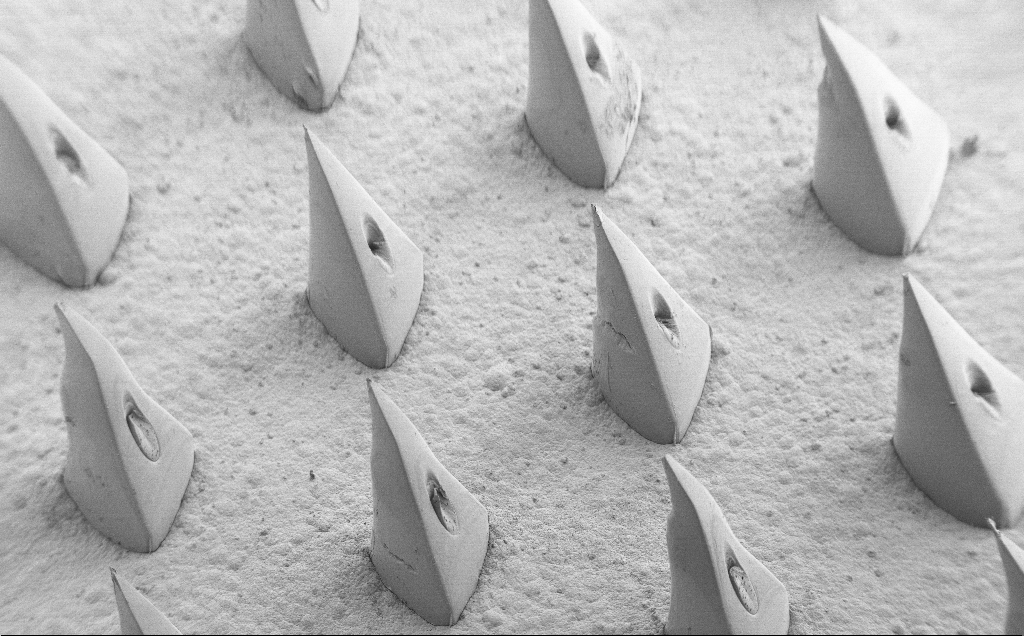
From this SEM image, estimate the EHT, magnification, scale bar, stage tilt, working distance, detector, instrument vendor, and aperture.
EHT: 5 kV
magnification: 0.075 K X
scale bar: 200000 nm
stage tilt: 40°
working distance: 8 mm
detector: SE2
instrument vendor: Zeiss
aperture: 30 µm